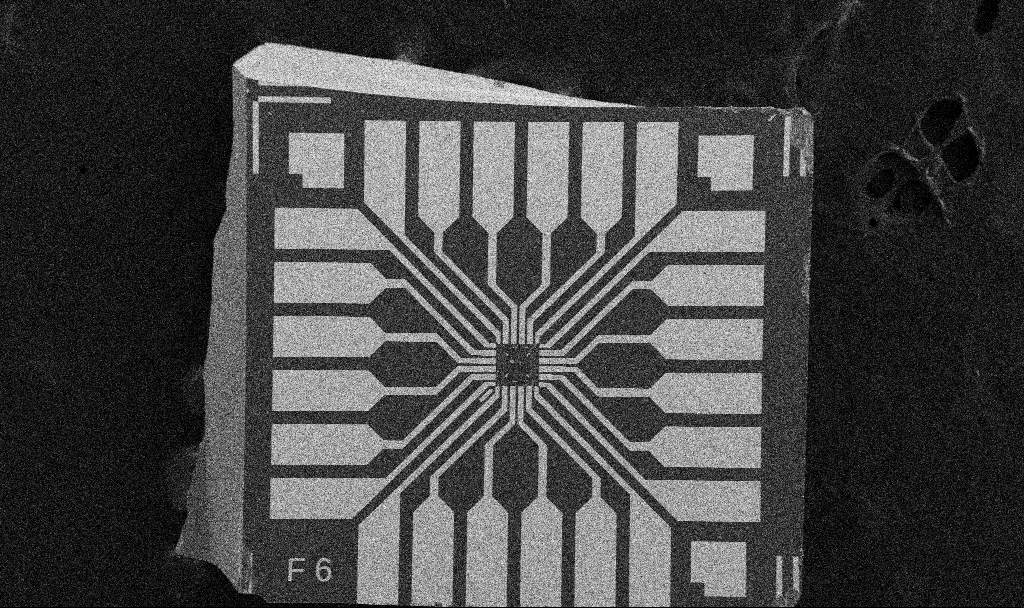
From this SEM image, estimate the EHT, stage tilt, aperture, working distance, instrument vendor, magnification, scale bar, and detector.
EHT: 5 kV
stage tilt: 0°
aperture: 30 µm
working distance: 7.6 mm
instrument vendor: Zeiss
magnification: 0.1 K X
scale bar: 200000 nm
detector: SE2